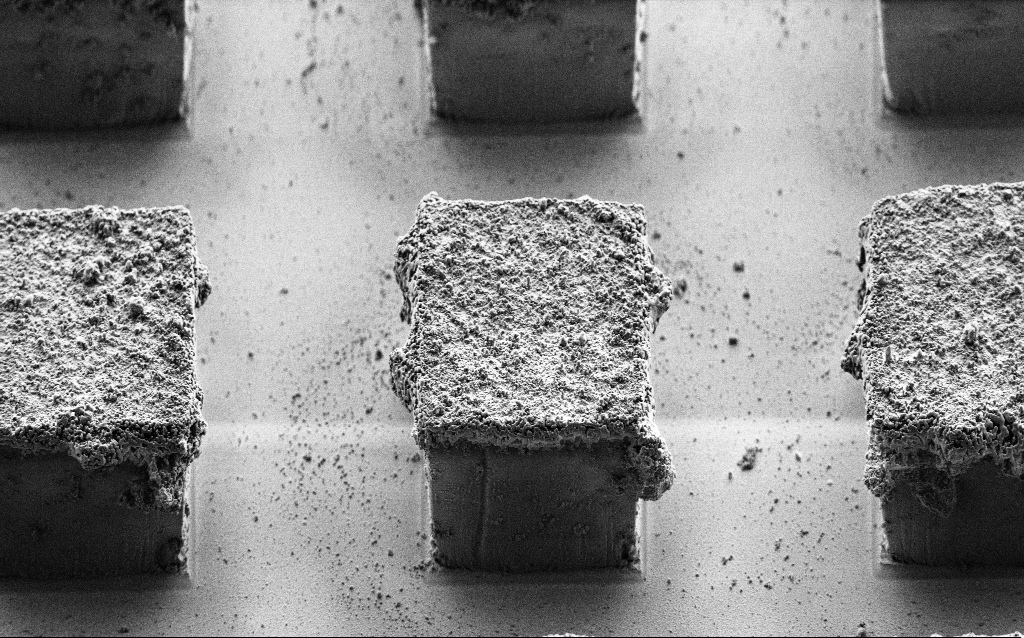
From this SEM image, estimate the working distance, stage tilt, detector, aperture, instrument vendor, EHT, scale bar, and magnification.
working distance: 7.3 mm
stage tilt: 45°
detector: SE2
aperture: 30 µm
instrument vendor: Zeiss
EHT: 1.5 kV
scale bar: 20000 nm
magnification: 1.65 K X